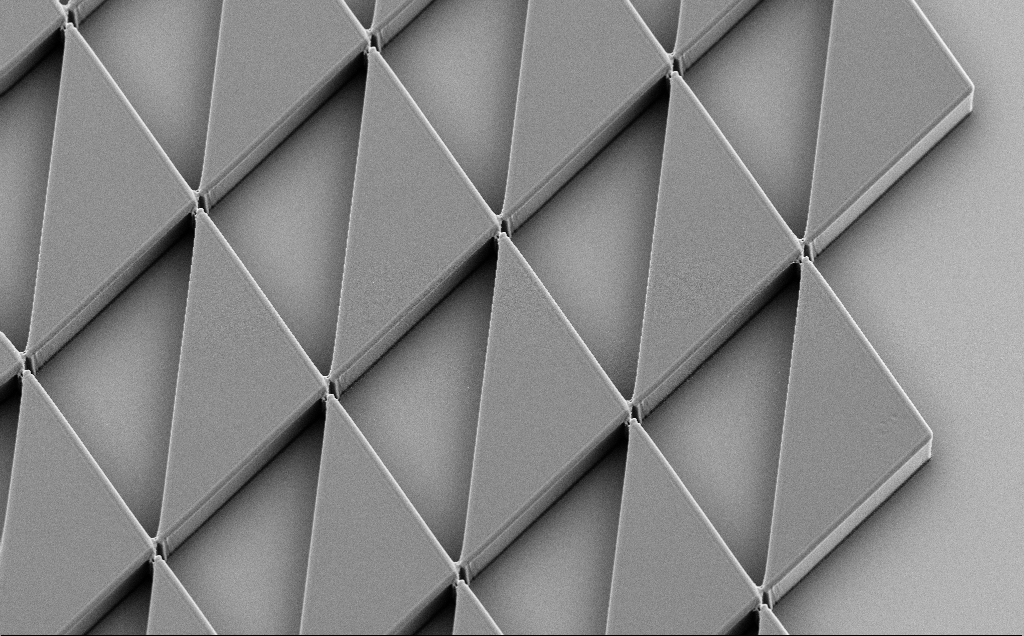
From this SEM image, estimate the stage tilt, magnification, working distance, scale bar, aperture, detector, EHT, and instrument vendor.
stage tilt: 30°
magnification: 1.58 K X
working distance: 11 mm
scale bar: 10000 nm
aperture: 30 µm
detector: SE2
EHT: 5 kV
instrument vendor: Zeiss